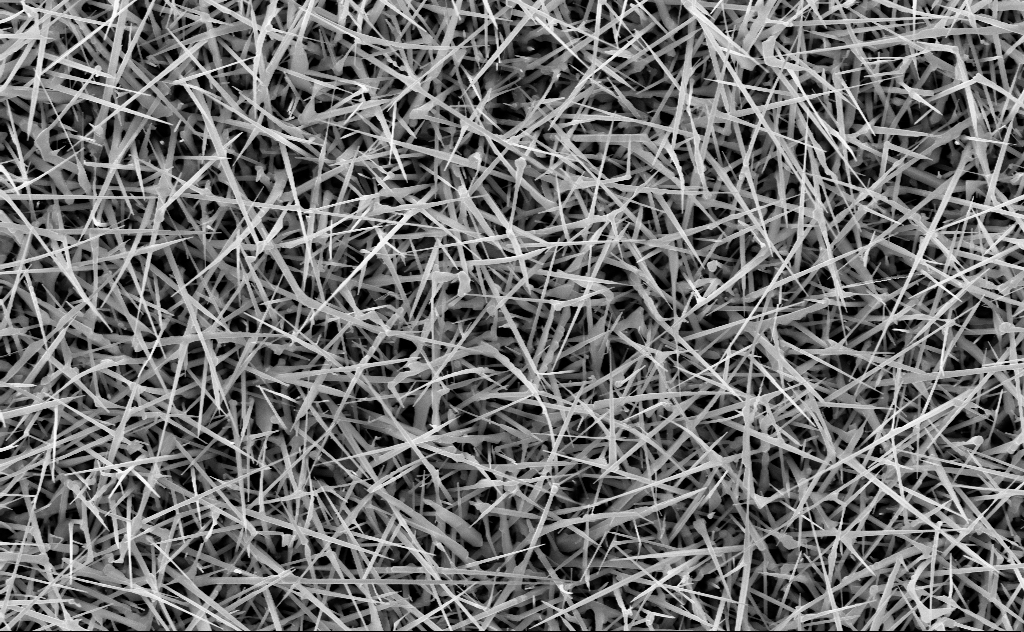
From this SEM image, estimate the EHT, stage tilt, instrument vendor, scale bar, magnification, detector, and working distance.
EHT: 10 kV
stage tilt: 0°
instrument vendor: Zeiss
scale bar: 1000 nm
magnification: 20 K X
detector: InLens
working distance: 10 mm